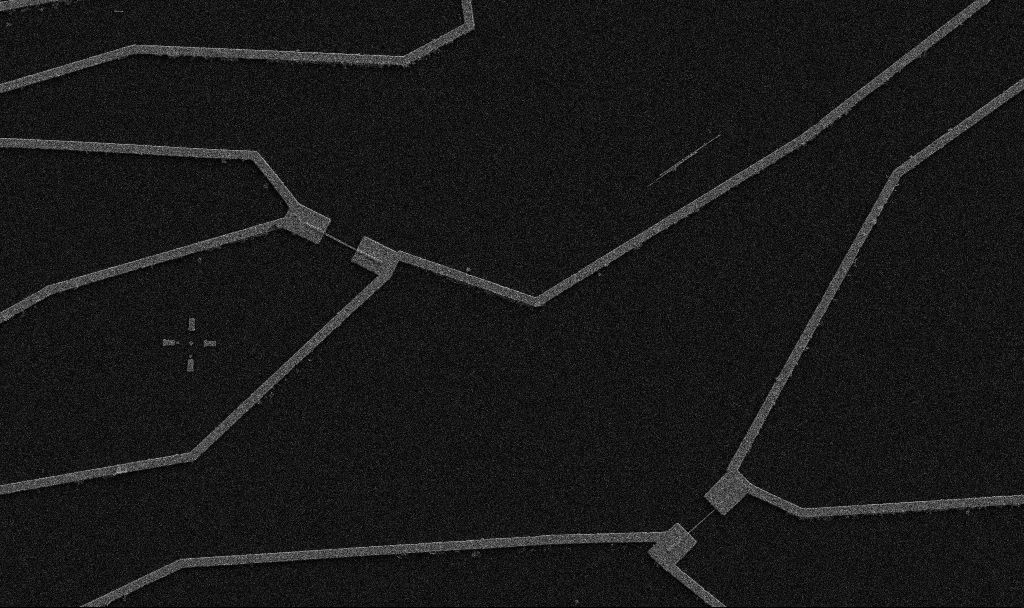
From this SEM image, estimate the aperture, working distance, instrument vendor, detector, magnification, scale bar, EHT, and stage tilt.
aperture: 30 µm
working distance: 10.7 mm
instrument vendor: Zeiss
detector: SE2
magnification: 5 K X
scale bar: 10000 nm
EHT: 5 kV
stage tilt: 0°